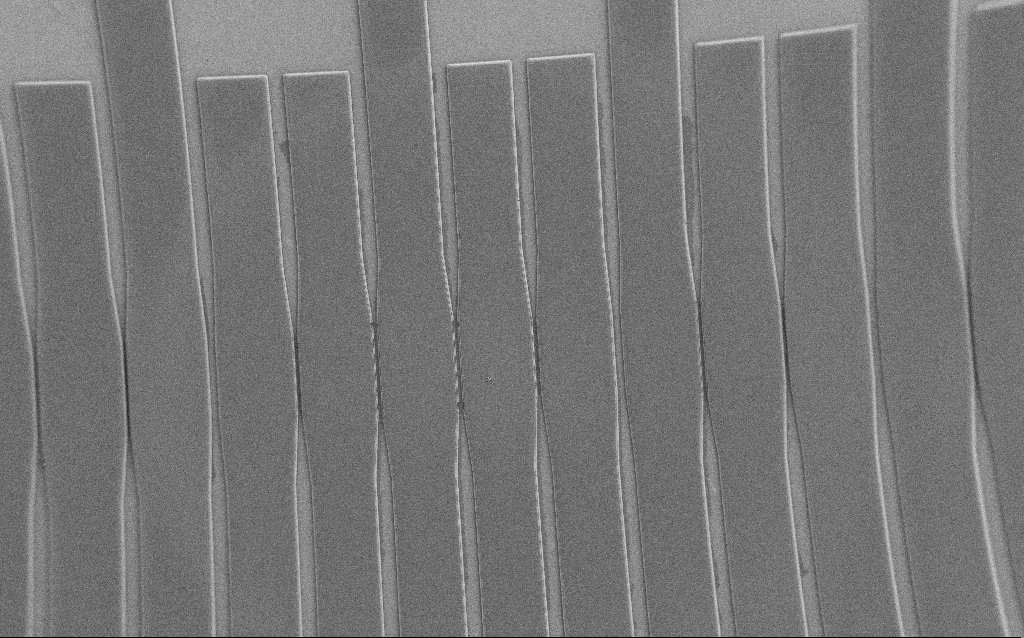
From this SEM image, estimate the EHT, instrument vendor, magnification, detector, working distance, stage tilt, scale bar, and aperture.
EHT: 1 kV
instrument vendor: Zeiss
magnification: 0.277 K X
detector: SE2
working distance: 6 mm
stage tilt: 0°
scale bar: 100000 nm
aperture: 30 µm